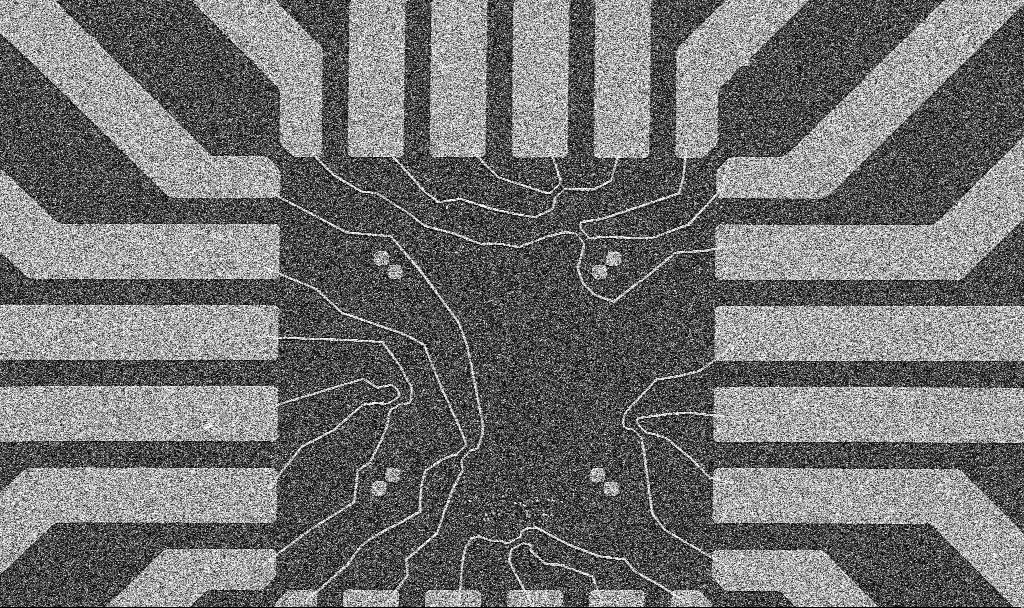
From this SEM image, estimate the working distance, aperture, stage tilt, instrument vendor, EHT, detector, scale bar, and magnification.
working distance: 10.7 mm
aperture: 30 µm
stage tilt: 0°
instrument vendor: Zeiss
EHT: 10 kV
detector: SE2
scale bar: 20000 nm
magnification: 1 K X